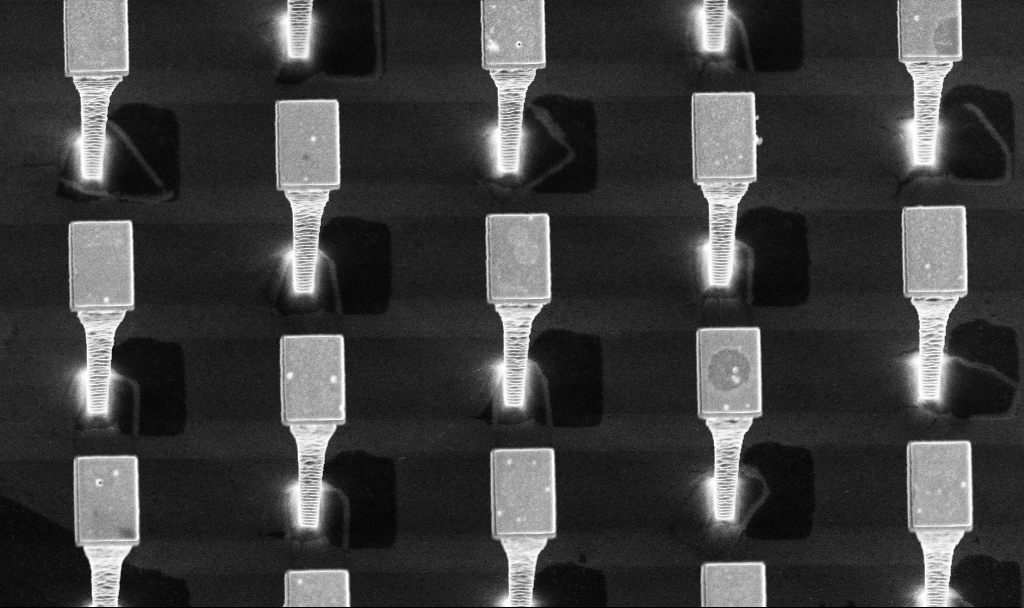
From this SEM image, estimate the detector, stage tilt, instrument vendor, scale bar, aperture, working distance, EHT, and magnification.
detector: InLens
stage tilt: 20°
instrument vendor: Zeiss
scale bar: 2000 nm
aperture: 30 µm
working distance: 4.2 mm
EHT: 5 kV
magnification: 7.69 K X